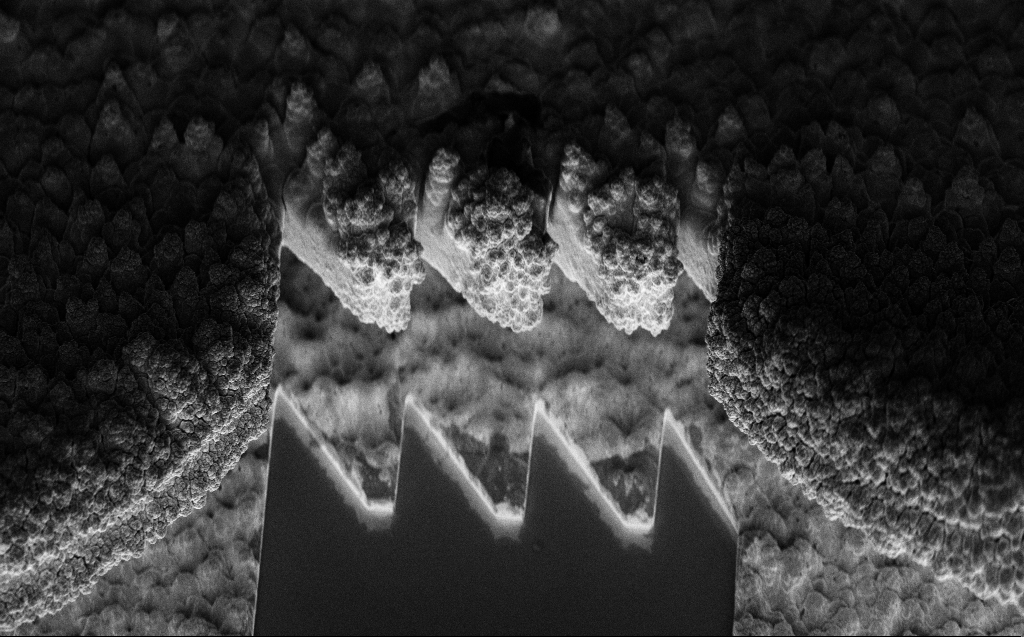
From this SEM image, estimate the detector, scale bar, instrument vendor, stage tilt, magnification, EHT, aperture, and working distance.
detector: InLens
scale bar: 10000 nm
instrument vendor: Zeiss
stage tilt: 45.1°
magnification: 4.2 K X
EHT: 5 kV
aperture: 30 µm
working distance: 9 mm